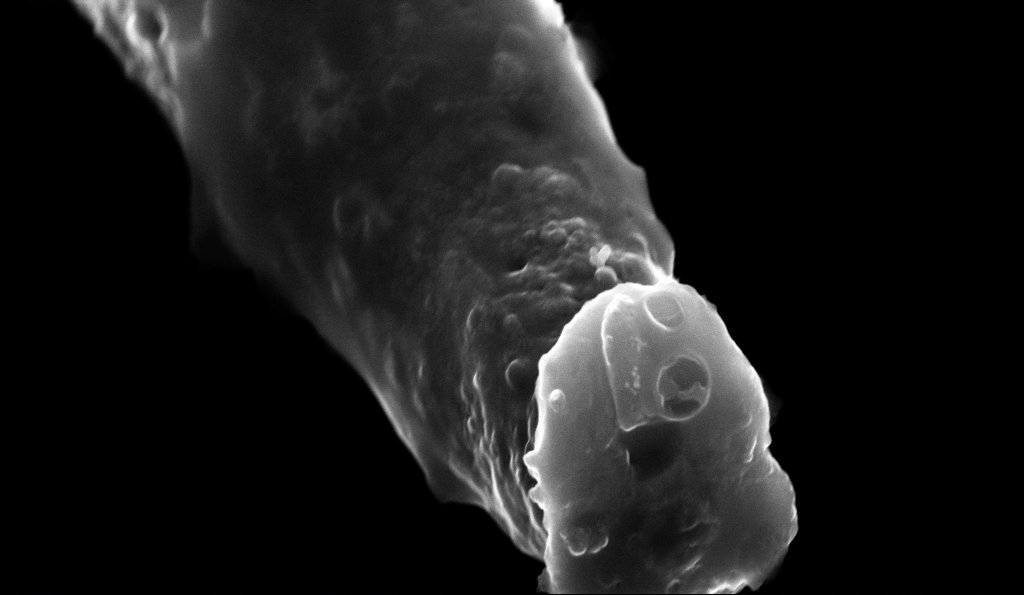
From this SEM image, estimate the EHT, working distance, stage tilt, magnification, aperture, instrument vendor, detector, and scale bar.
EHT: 10 kV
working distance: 2.2 mm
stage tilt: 56°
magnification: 60.58 K X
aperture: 30 µm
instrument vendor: Zeiss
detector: InLens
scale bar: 1000 nm